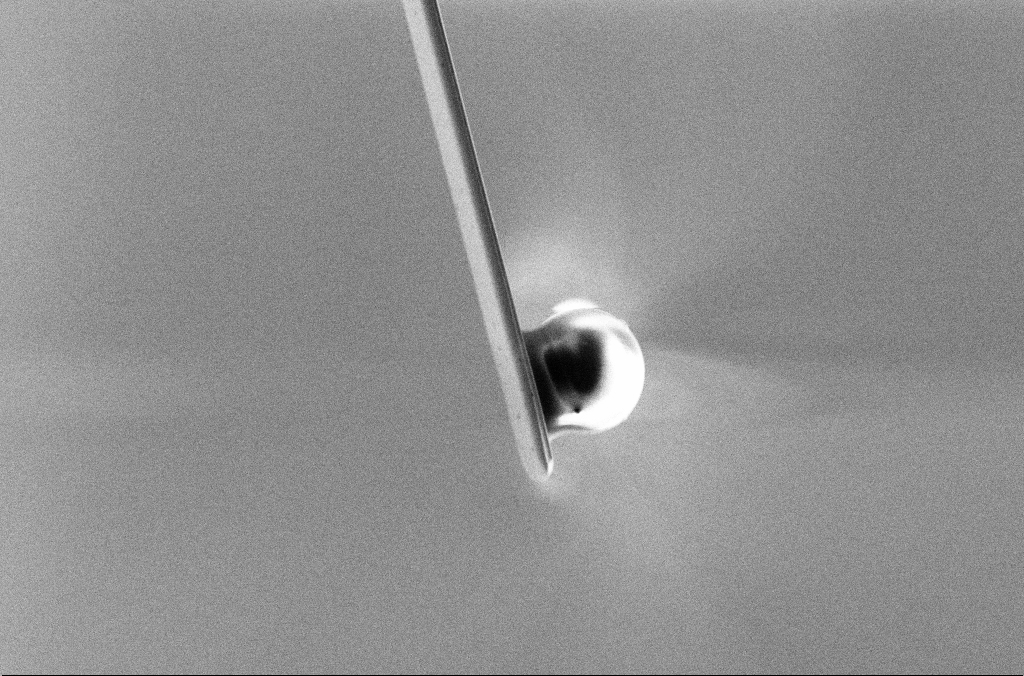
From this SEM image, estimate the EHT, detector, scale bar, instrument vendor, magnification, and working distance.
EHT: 5 kV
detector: SE2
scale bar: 10000 nm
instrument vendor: Zeiss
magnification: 2.5 K X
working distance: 5.3 mm